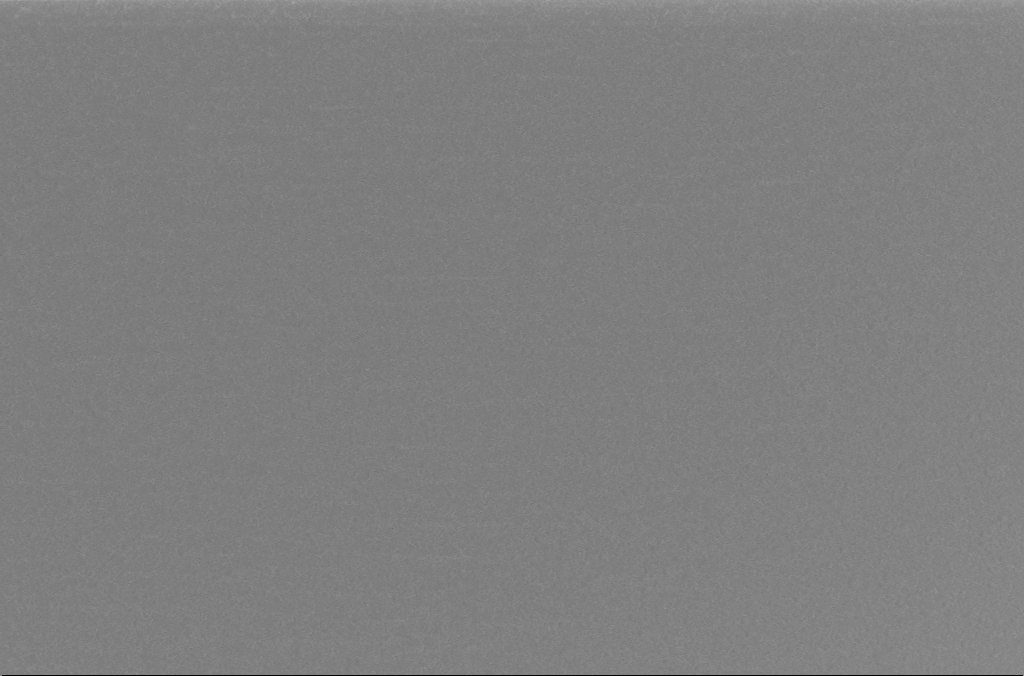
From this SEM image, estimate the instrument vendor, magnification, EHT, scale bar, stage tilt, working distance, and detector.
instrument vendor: Zeiss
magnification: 5 K X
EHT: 5 kV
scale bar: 10000 nm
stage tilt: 0°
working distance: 3 mm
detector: InLens